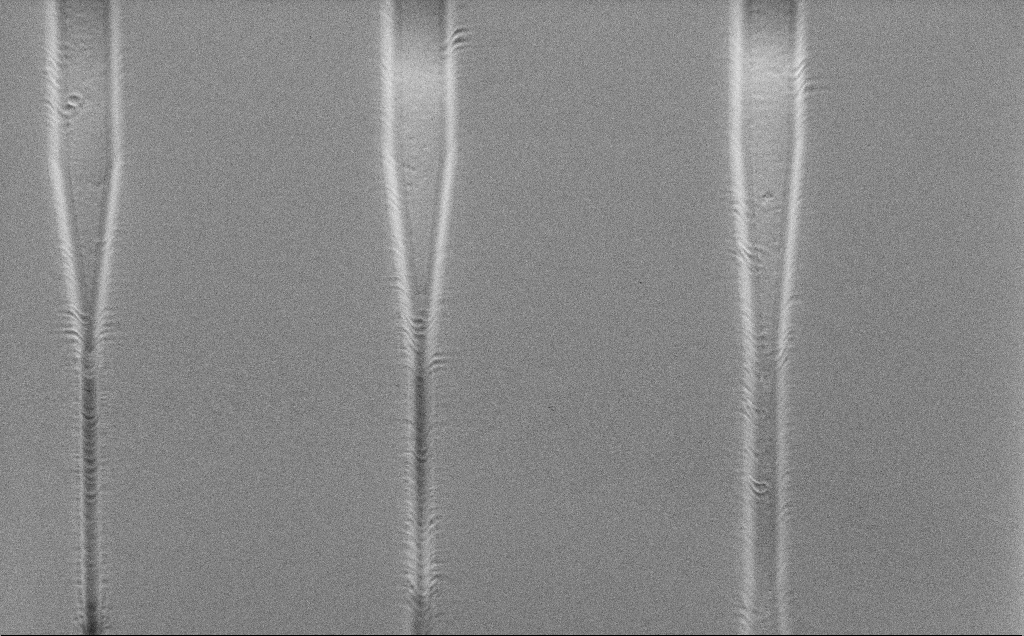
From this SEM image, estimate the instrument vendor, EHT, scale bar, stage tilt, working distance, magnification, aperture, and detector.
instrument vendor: Zeiss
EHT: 1 kV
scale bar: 20000 nm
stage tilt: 45°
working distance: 7 mm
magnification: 1.17 K X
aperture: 30 µm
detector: SE2